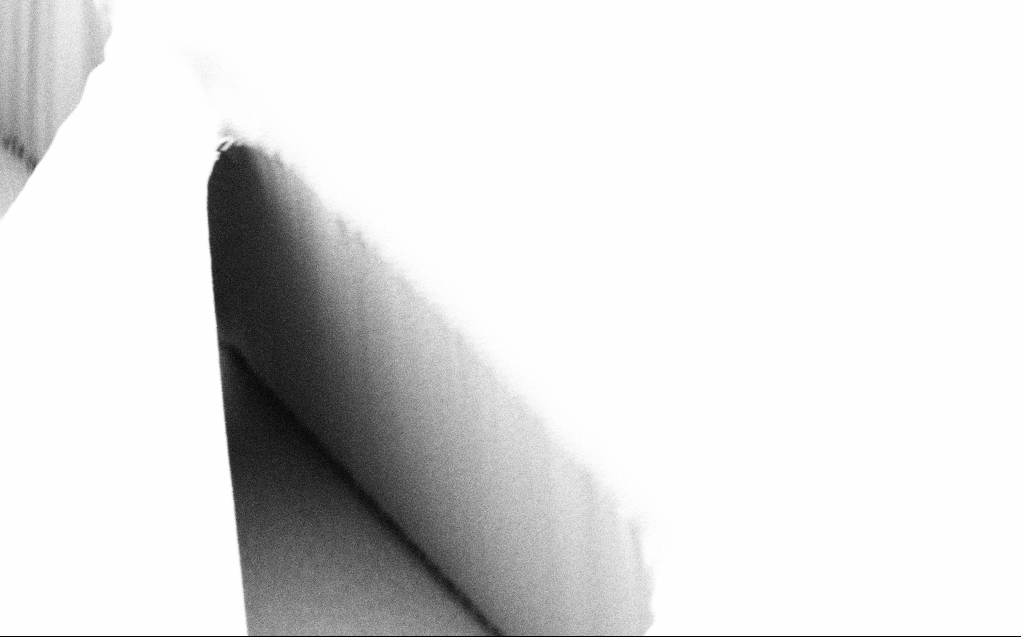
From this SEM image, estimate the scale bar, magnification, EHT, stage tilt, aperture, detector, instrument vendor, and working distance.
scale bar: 10000 nm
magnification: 5.06 K X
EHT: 1 kV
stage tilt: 45°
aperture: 30 µm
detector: SE2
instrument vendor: Zeiss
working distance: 6 mm